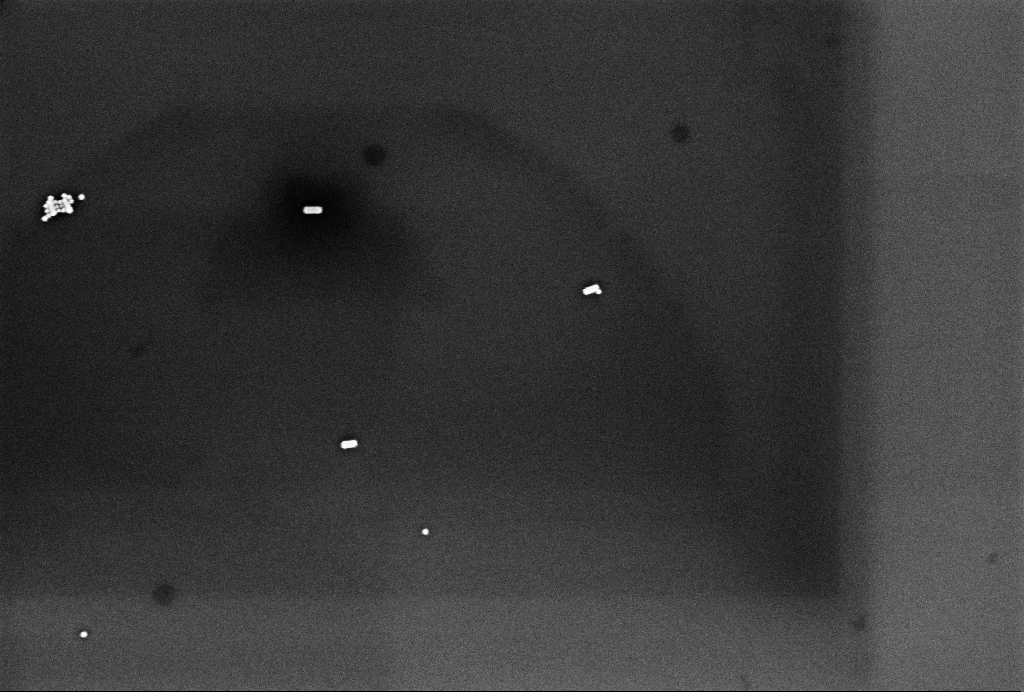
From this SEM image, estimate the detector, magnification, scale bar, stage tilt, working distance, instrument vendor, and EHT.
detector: InLens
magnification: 80.44 K X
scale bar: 200 nm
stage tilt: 0°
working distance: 3.3 mm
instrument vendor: Zeiss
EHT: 2 kV